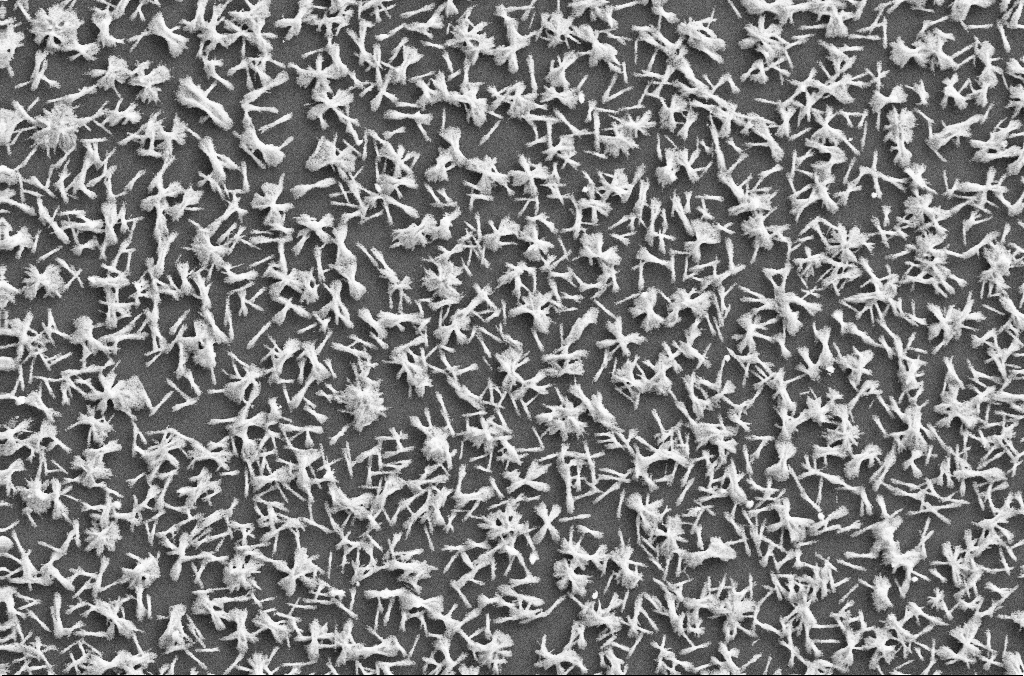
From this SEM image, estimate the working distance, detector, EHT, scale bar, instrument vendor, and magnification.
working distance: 2.8 mm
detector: SE2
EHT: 20 kV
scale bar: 10000 nm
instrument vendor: Zeiss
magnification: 2 K X